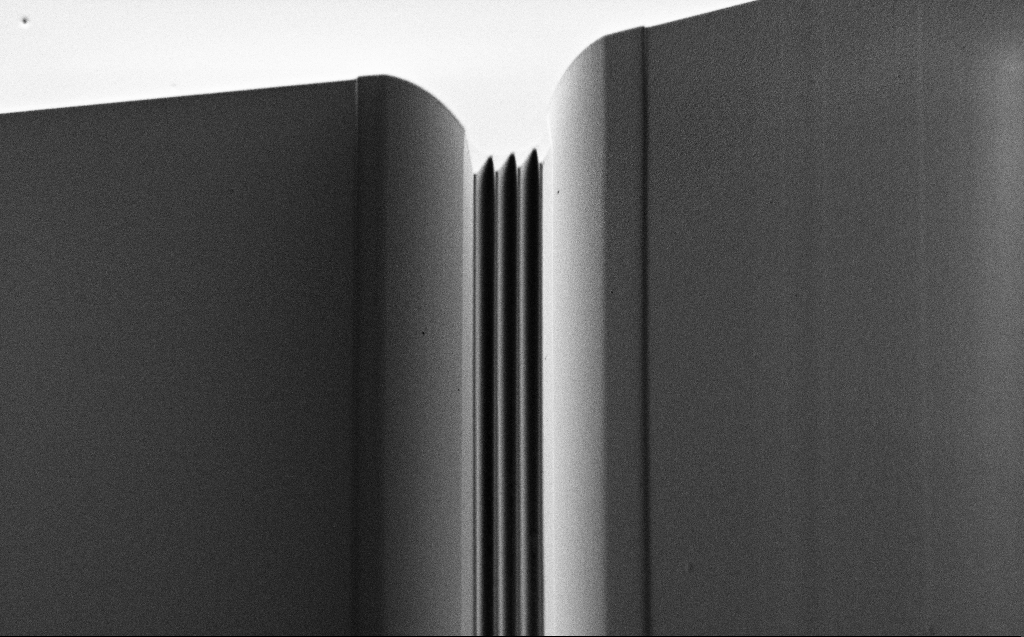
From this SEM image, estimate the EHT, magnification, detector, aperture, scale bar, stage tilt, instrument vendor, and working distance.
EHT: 0.9 kV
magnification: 0.73 K X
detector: SE2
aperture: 30 µm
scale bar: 20000 nm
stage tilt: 45°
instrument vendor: Zeiss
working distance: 6 mm